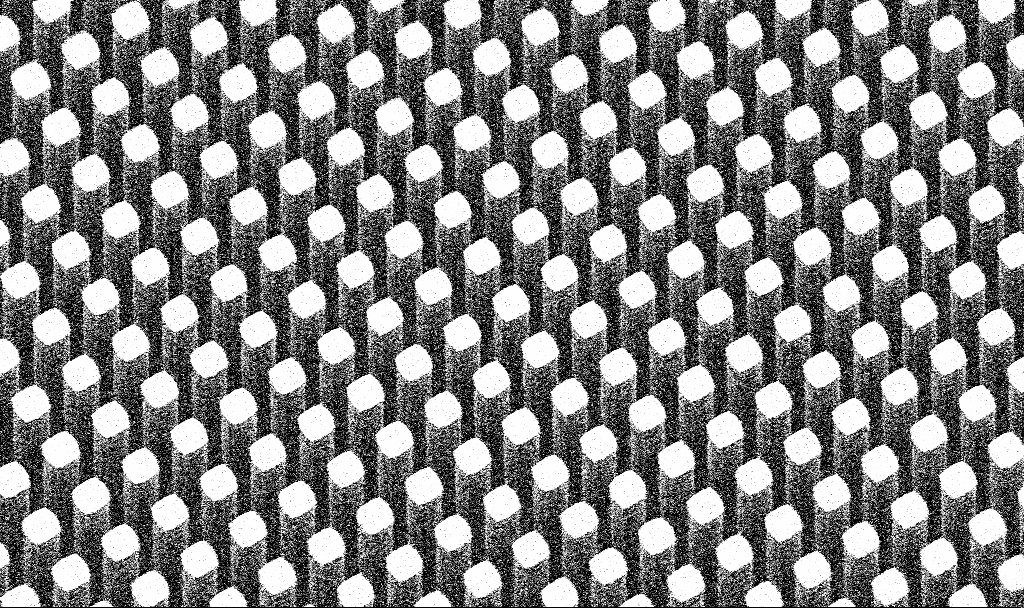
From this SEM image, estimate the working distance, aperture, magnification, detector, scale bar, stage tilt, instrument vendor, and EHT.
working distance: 7.5 mm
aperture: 30 µm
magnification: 5.24 K X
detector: SE2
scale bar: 10000 nm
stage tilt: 45°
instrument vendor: Zeiss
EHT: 5 kV